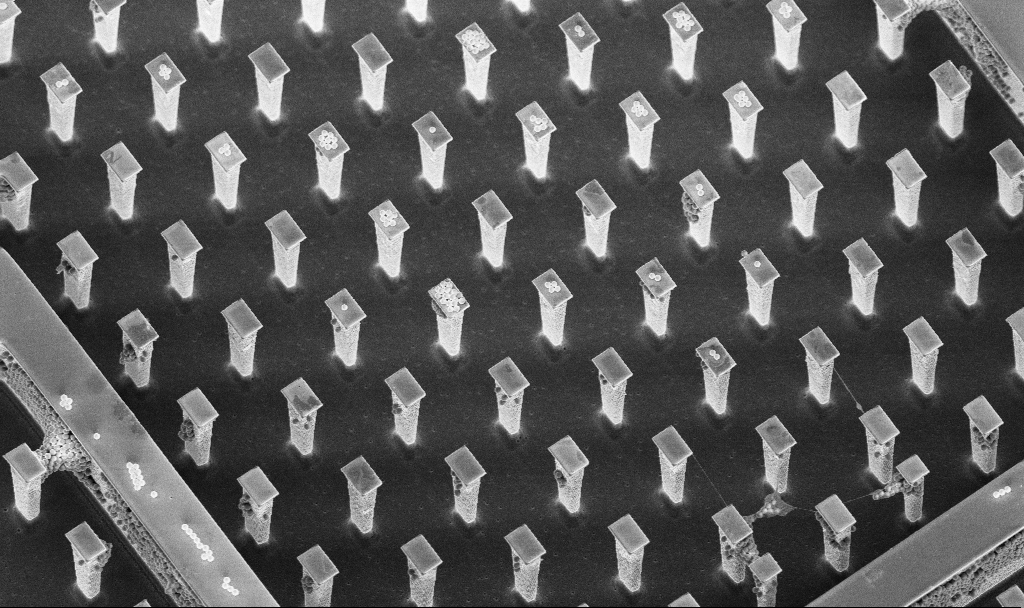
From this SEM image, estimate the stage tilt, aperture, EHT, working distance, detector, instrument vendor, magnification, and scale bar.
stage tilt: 20°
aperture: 30 µm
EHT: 5 kV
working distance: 4.8 mm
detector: InLens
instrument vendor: Zeiss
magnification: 3.17 K X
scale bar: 10000 nm